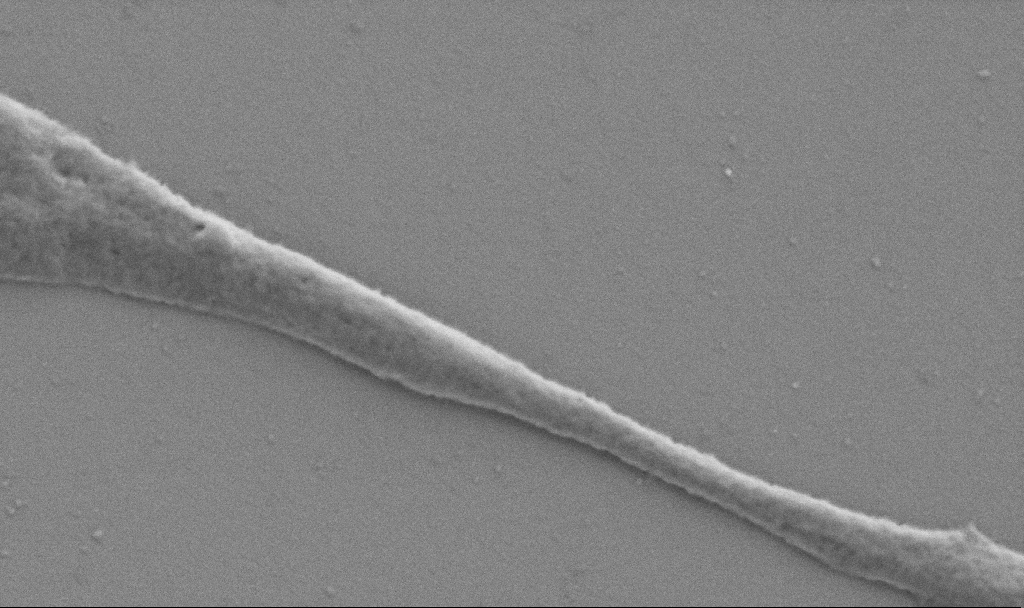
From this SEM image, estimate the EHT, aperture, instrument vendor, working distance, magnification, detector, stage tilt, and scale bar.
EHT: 0.9 kV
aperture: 30 µm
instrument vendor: Zeiss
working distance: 6.9 mm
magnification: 50 K X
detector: SE2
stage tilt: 0°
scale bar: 1000 nm